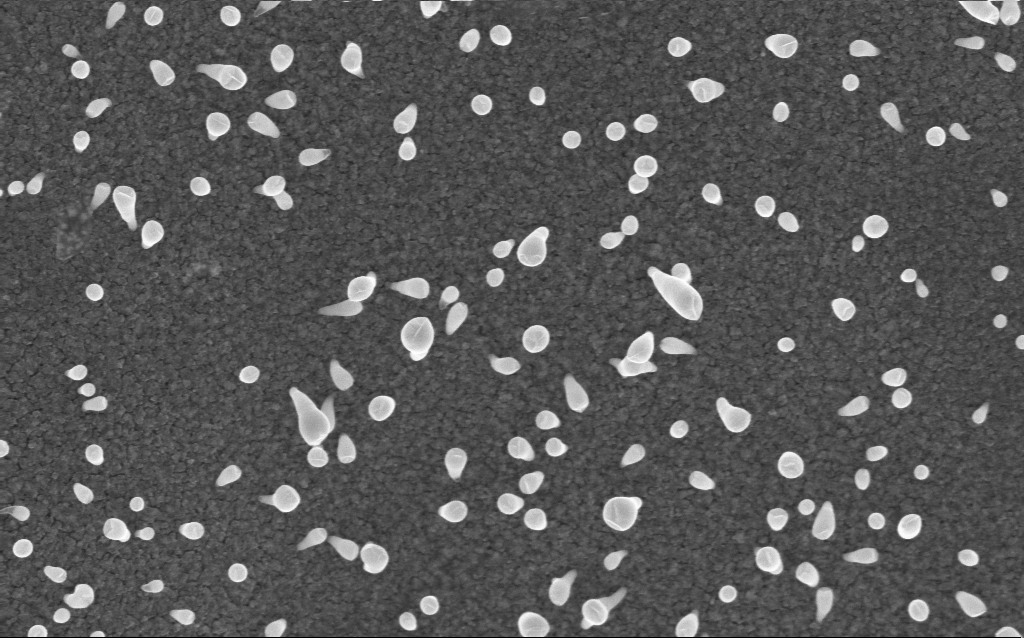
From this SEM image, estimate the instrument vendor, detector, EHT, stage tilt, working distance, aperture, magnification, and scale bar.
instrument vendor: Zeiss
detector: InLens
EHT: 5 kV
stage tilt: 0°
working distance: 4 mm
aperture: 30 µm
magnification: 80 K X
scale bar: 200 nm